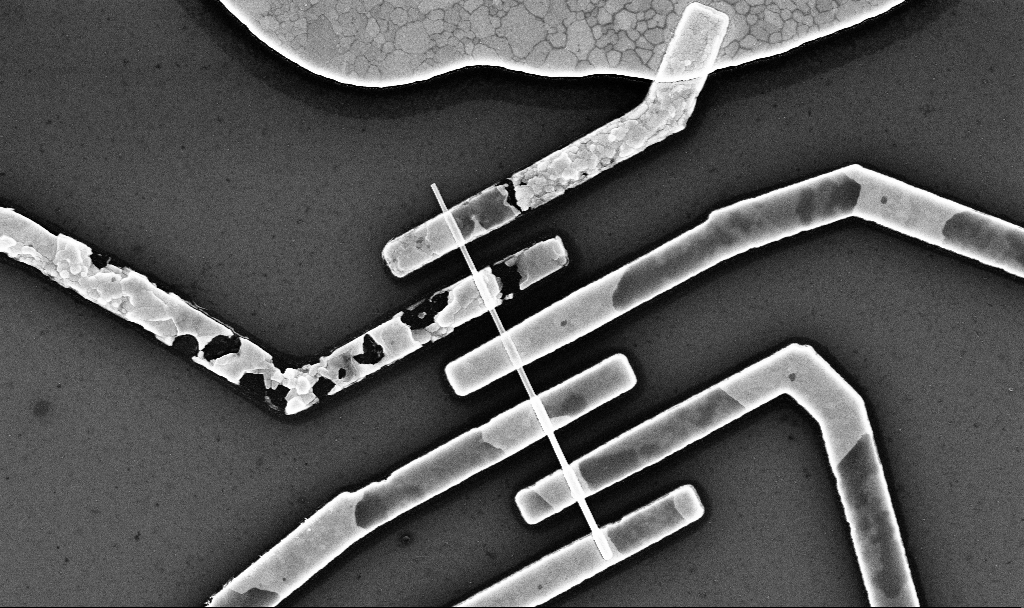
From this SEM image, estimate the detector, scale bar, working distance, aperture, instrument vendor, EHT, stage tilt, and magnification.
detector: InLens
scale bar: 2000 nm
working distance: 6.8 mm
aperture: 30 µm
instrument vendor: Zeiss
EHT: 10 kV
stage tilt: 0°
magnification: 23.18 K X